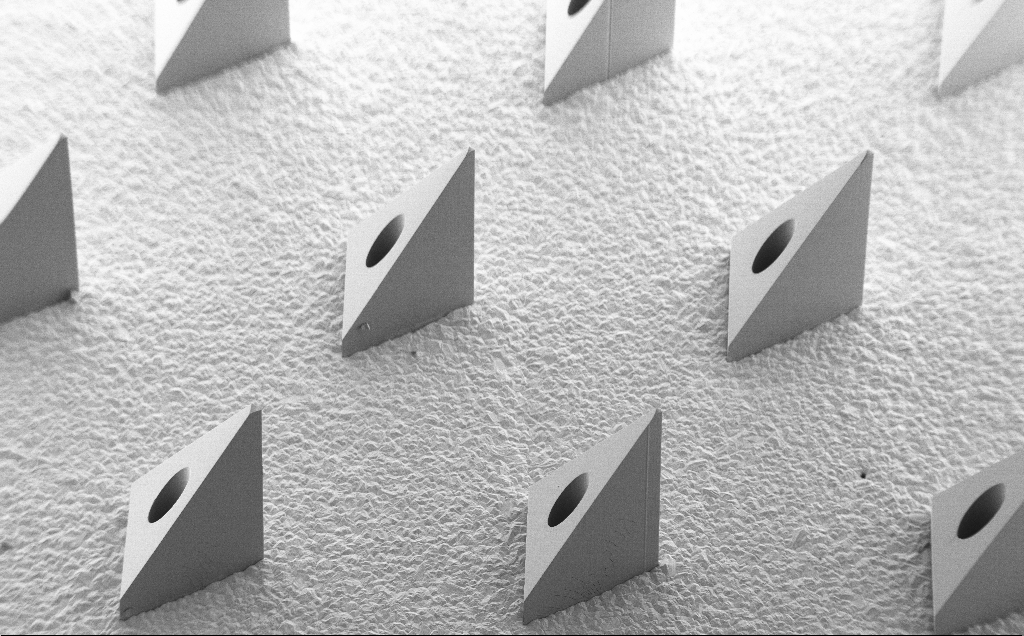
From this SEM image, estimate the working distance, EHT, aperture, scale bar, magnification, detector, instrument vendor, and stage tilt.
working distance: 9 mm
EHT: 5 kV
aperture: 30 µm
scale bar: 200000 nm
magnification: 0.091 K X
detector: SE2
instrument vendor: Zeiss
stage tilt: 40°